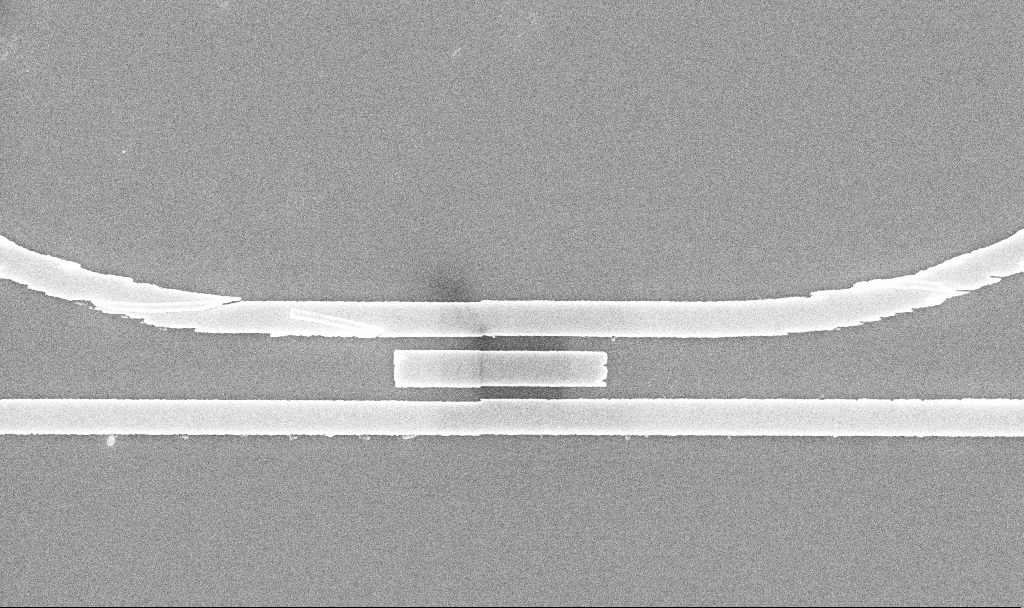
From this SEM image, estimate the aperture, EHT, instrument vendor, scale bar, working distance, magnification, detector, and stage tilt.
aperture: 30 µm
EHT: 5 kV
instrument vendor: Zeiss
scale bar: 1000 nm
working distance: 10.3 mm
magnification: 25.78 K X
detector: InLens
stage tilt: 0°